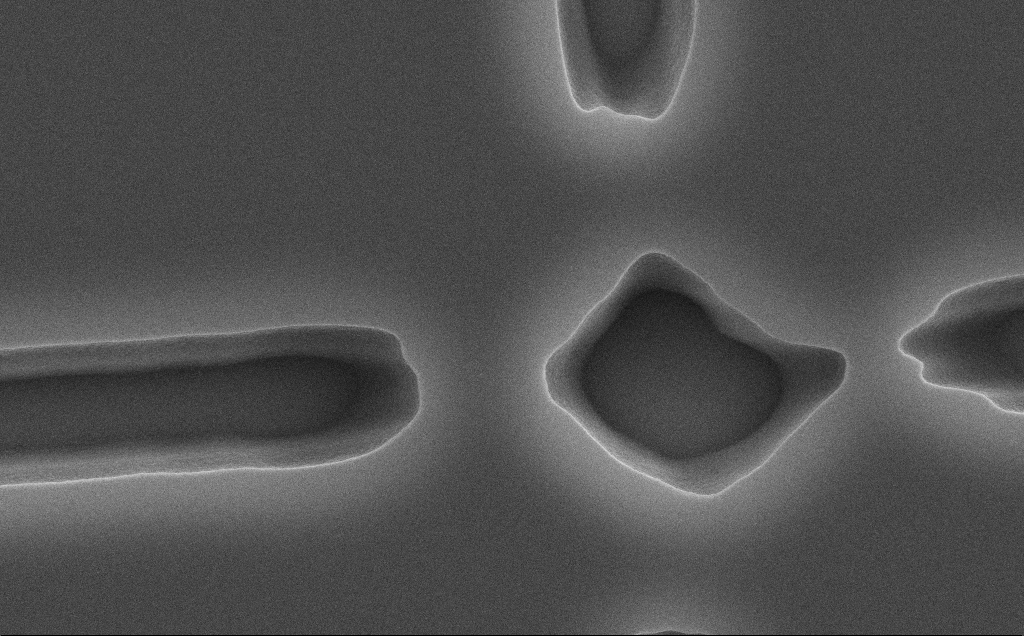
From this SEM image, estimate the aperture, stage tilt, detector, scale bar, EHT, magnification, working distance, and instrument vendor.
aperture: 30 µm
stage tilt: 0°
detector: SE2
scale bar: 2000 nm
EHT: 10 kV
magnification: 20.68 K X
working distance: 13 mm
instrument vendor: Zeiss